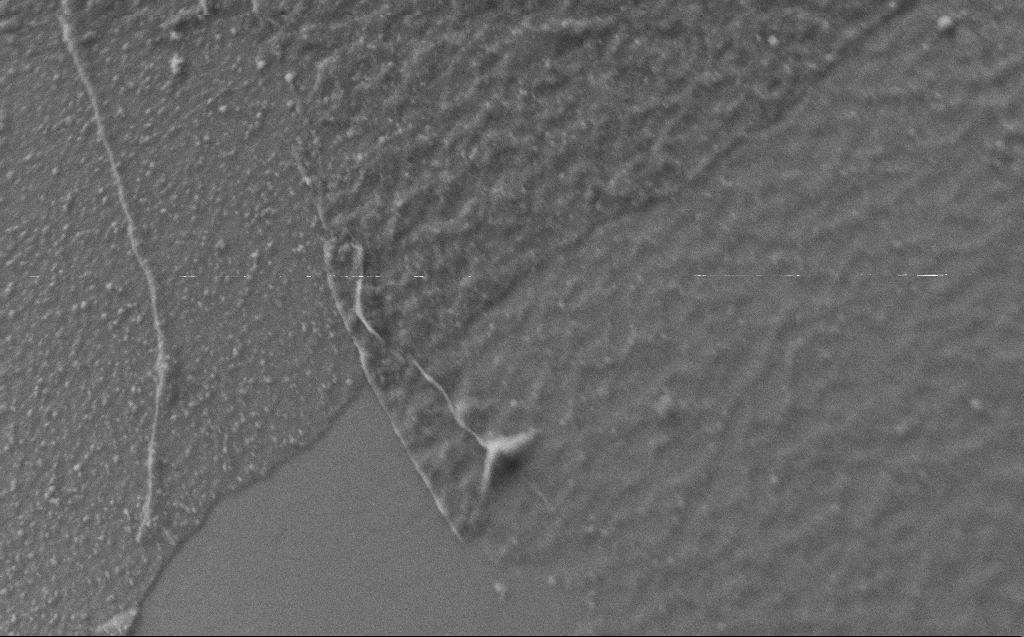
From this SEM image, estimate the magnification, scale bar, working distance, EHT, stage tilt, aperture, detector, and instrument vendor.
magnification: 50 K X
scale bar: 1000 nm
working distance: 3 mm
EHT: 2 kV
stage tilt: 45°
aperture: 30 µm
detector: SE2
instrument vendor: Zeiss